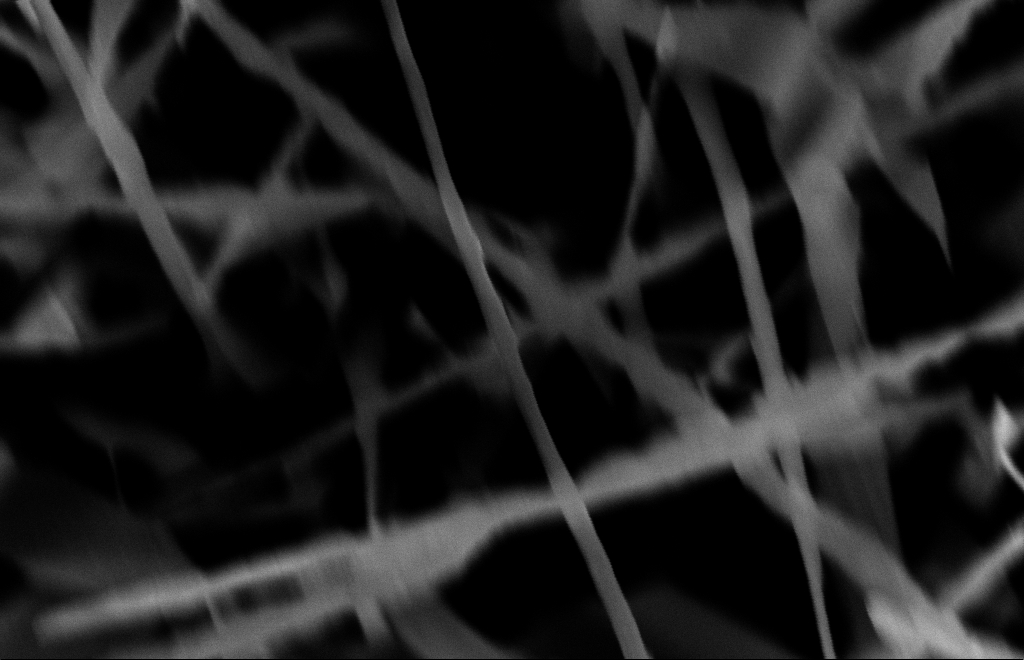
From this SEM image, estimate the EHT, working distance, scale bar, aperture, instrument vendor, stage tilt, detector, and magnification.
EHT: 10 kV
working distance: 15 mm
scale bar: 100 nm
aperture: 30 µm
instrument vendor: Zeiss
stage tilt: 0°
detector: InLens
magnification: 80 K X